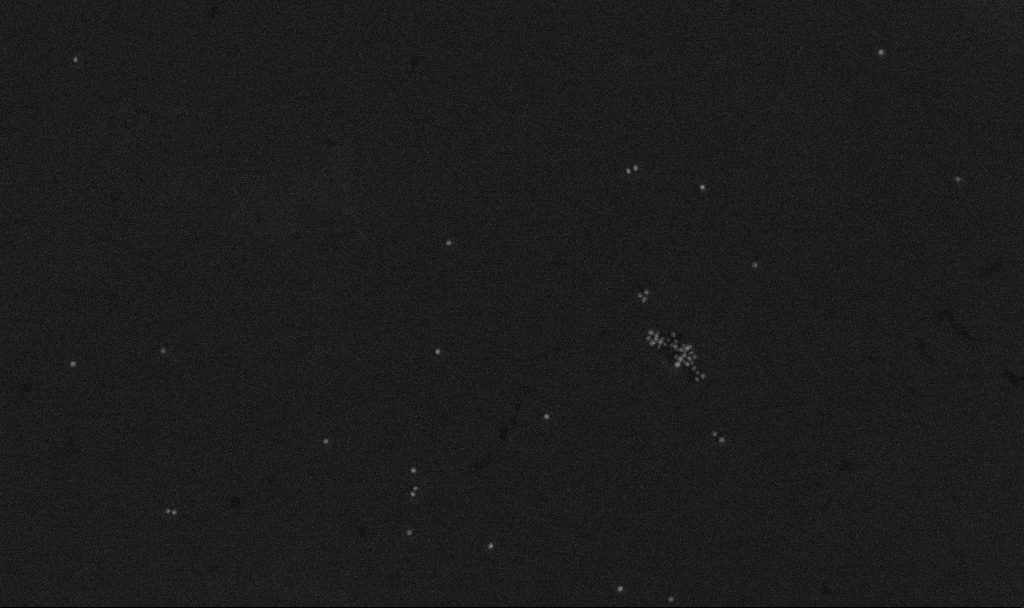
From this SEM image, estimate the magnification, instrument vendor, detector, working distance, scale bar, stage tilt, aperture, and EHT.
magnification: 100 K X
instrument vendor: Zeiss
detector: InLens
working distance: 3.3 mm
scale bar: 200 nm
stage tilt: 0°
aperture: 30 µm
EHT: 10 kV